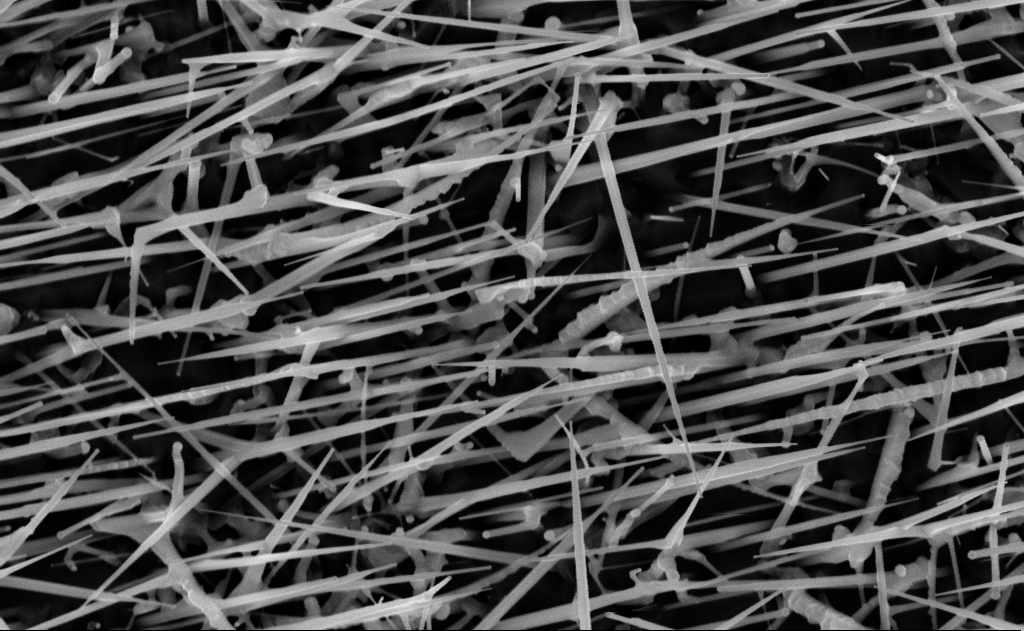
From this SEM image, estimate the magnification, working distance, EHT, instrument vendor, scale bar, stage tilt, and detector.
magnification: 40 K X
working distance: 15 mm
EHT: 10 kV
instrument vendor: Zeiss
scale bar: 1000 nm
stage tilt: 0°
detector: InLens